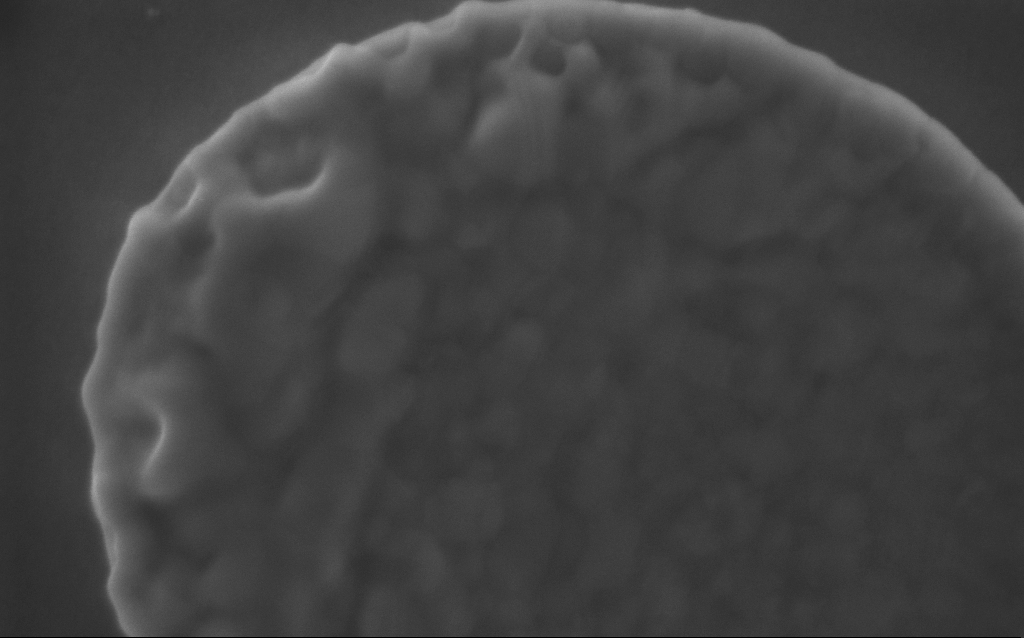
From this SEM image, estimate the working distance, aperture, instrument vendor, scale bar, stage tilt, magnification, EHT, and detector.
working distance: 3 mm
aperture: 30 µm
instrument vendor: Zeiss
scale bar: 200 nm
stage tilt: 0°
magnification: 234 K X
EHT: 5 kV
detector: InLens